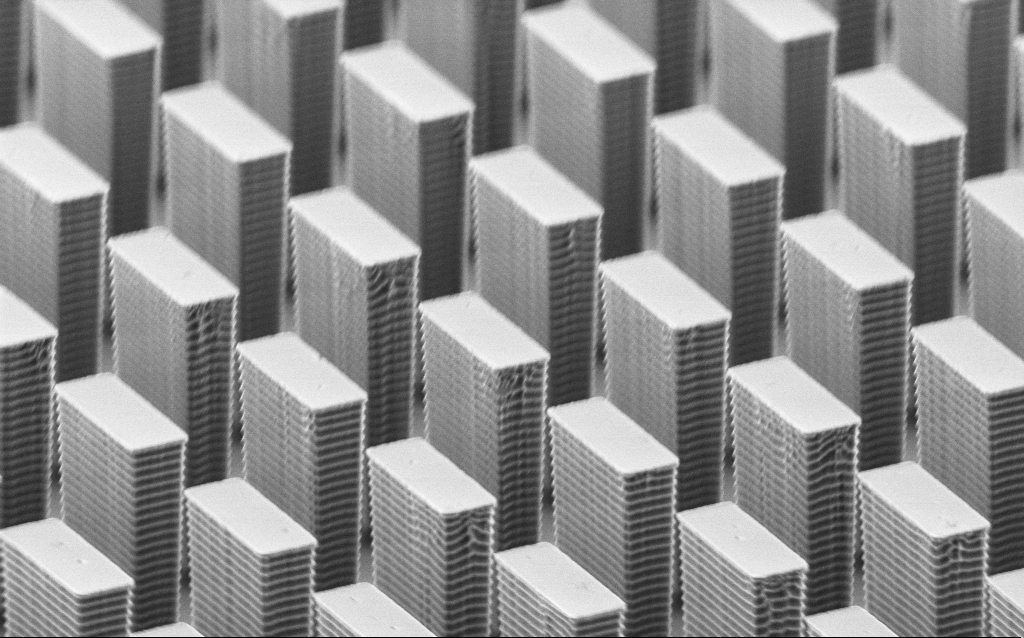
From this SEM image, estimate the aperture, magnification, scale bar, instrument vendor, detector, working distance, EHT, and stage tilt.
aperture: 30 µm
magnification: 13.16 K X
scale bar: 2000 nm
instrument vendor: Zeiss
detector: SE2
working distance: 9.8 mm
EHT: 3 kV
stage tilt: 67°